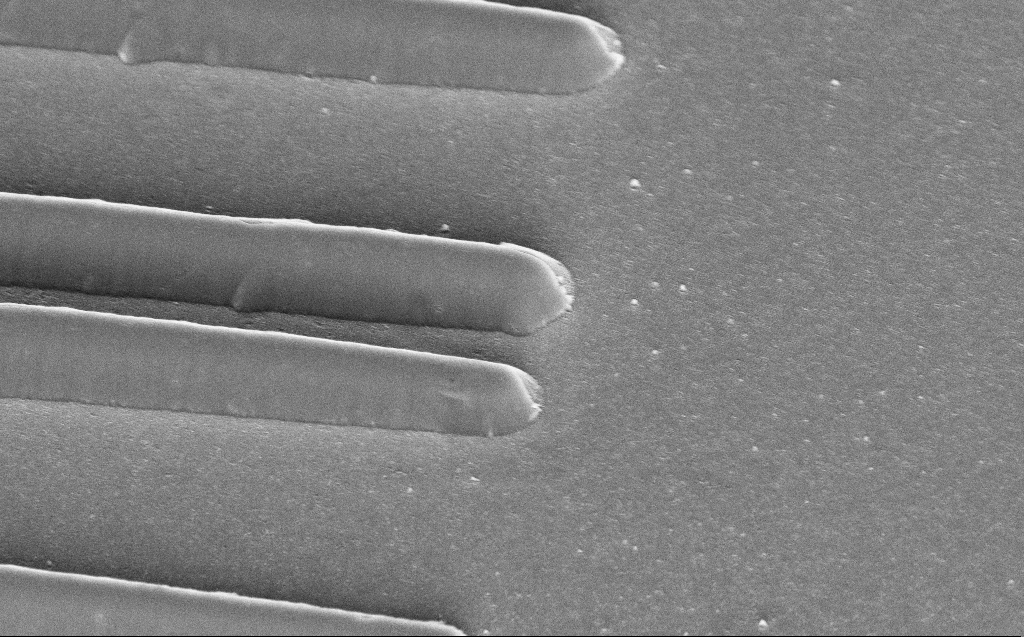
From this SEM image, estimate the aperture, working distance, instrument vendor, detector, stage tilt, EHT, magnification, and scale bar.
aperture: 30 µm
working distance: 10 mm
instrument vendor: Zeiss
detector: InLens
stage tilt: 45.1°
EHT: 5 kV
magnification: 10.67 K X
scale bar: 2000 nm